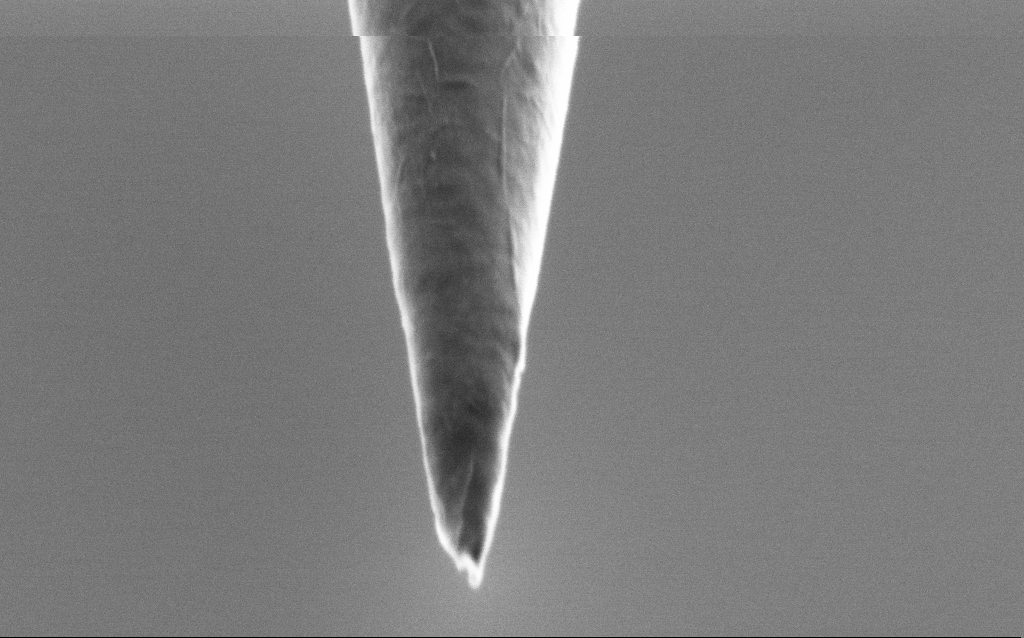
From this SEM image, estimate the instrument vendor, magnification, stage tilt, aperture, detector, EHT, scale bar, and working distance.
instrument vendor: Zeiss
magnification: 100 K X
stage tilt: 45°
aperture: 30 µm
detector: SE2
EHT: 1 kV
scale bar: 200 nm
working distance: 6 mm